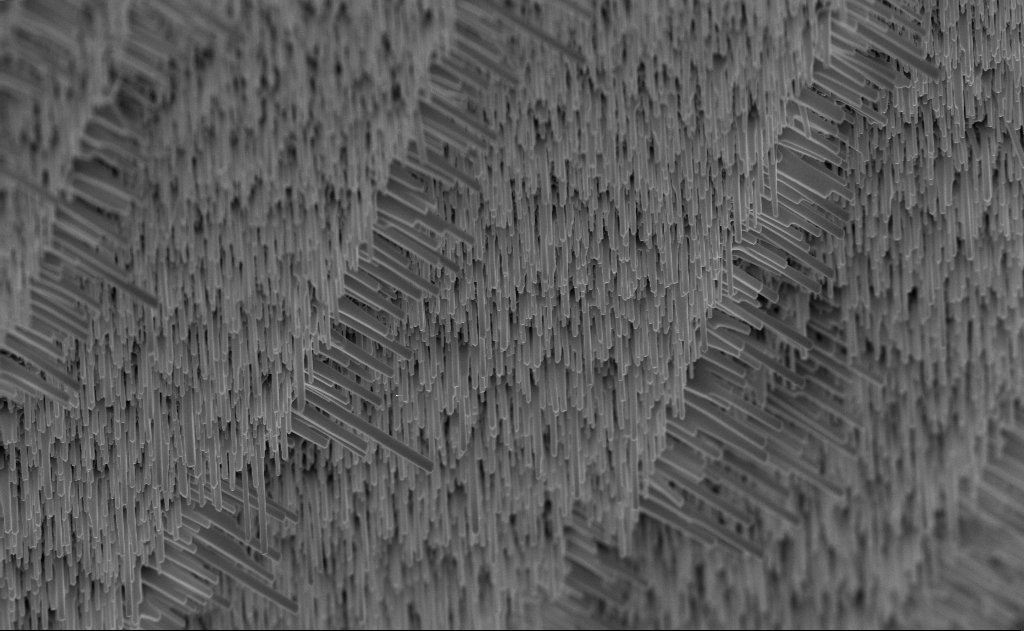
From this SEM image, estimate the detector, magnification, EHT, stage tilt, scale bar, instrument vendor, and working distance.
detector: InLens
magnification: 20 K X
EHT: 10 kV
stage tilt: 0°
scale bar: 2000 nm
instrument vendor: Zeiss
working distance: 6 mm